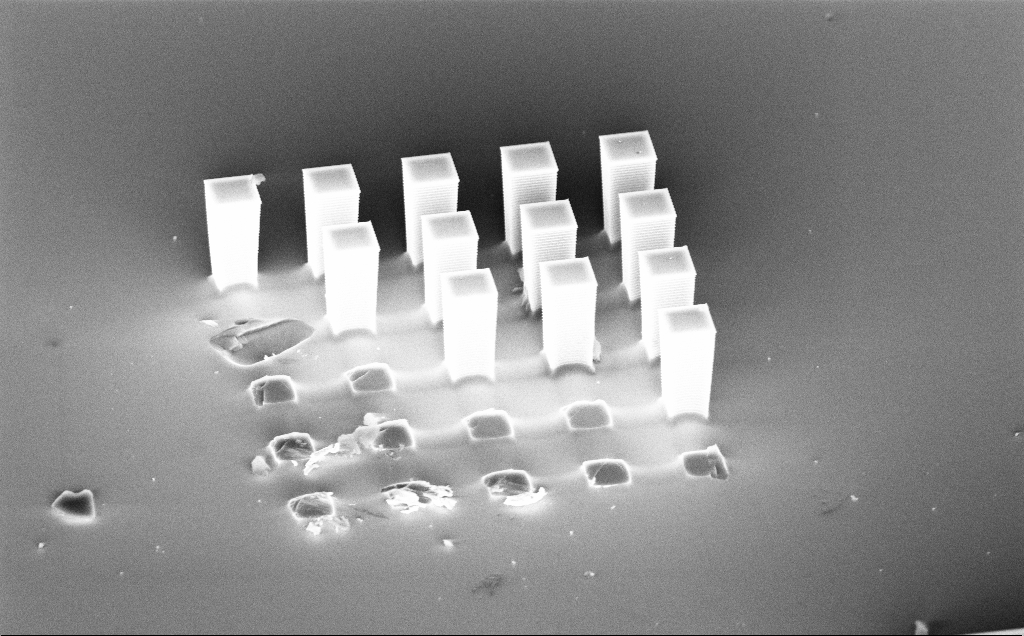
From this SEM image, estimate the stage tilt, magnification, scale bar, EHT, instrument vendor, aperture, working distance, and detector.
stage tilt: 54.6°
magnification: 3.73 K X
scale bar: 10000 nm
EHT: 10 kV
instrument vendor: Zeiss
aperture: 30 µm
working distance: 7 mm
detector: InLens